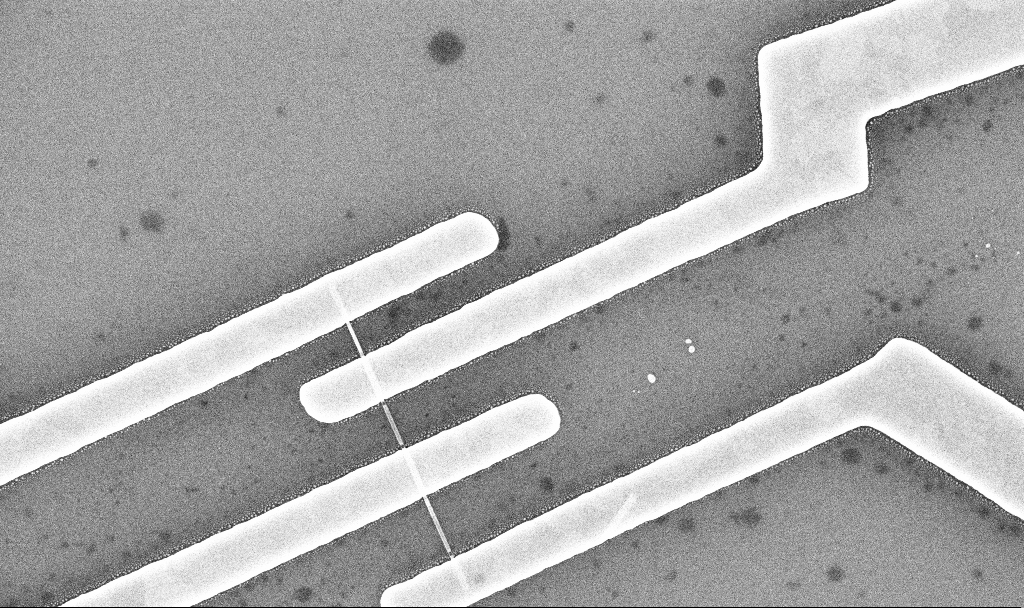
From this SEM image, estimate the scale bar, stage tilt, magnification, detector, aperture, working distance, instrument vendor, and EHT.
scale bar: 1000 nm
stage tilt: -0°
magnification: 42.61 K X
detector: InLens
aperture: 30 µm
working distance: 7 mm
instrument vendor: Zeiss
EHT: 10 kV